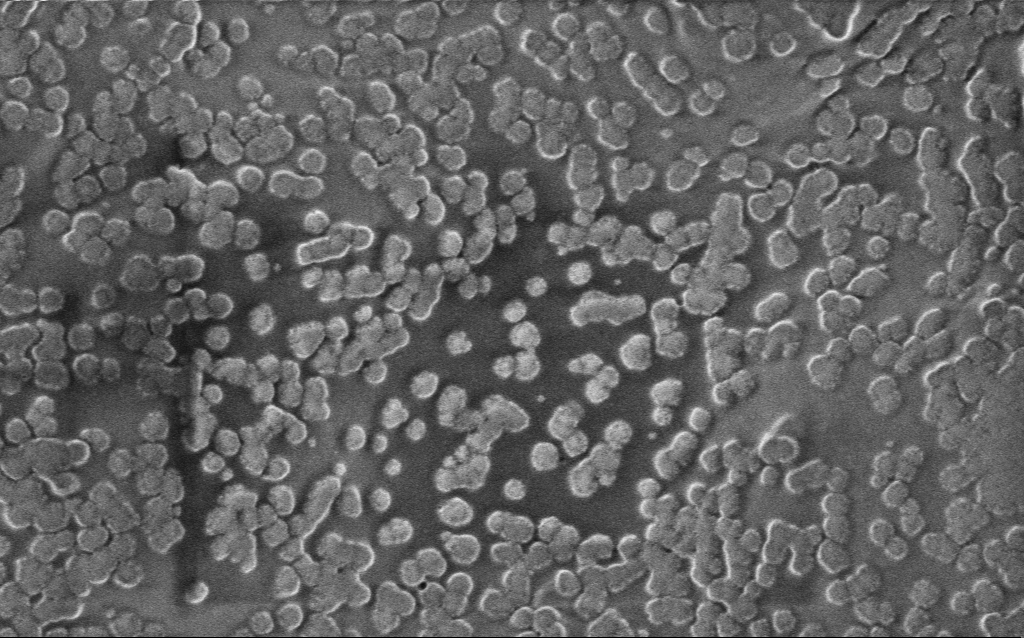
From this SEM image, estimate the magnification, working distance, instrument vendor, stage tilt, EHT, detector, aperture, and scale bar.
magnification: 54.98 K X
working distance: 4 mm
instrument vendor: Zeiss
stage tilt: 0°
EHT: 1 kV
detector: InLens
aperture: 30 µm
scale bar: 1000 nm